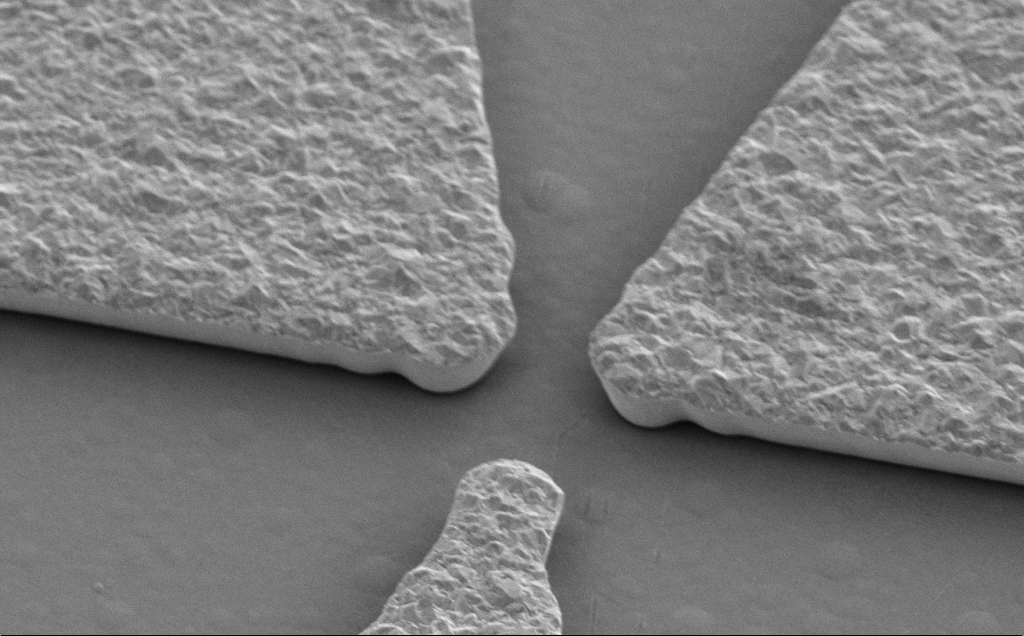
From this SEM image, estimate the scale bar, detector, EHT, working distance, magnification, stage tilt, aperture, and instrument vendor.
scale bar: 2000 nm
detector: SE2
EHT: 5 kV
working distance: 13 mm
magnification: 15.93 K X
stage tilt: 35°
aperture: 30 µm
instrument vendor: Zeiss